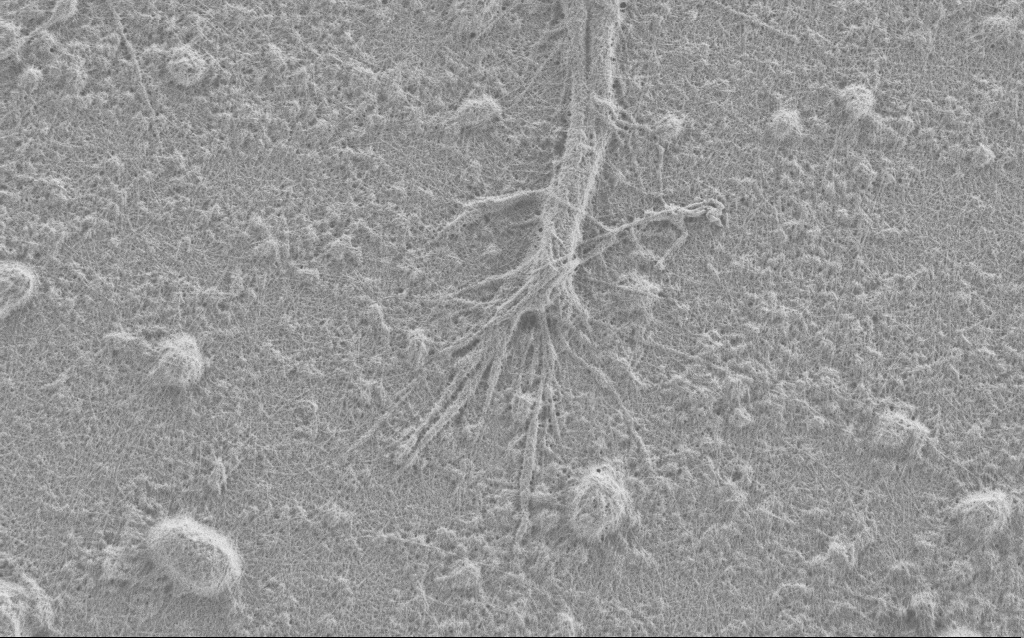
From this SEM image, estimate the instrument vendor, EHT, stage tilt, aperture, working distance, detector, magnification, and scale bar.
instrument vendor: Zeiss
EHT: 0.9 kV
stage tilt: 0°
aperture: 30 µm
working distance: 4 mm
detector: SE2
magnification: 10 K X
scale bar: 2000 nm